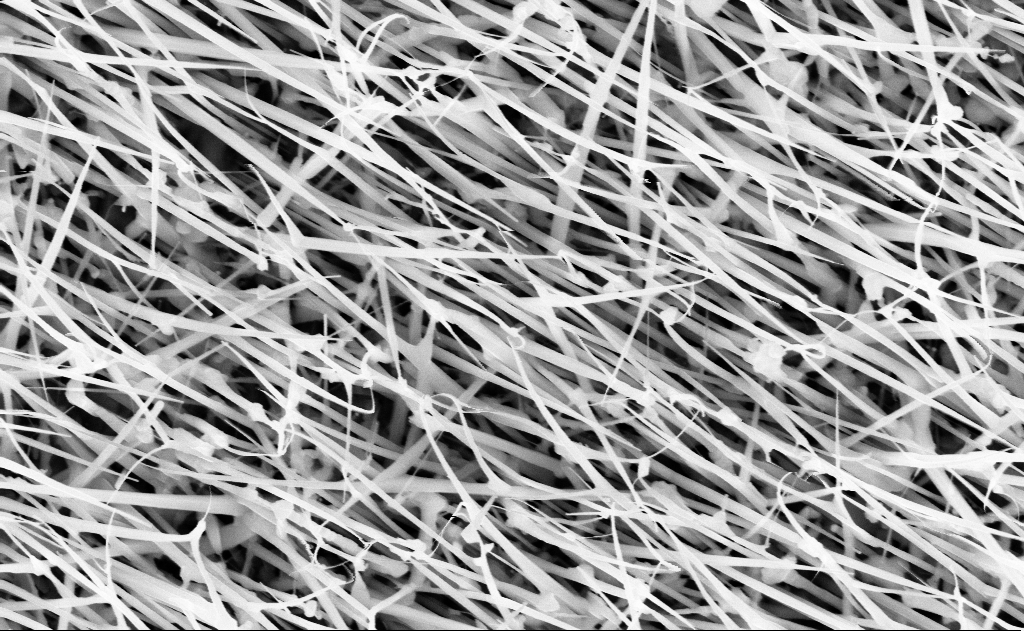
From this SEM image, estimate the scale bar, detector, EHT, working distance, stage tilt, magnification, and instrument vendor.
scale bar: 1000 nm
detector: InLens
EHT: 10 kV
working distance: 13 mm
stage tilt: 0°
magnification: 40 K X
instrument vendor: Zeiss